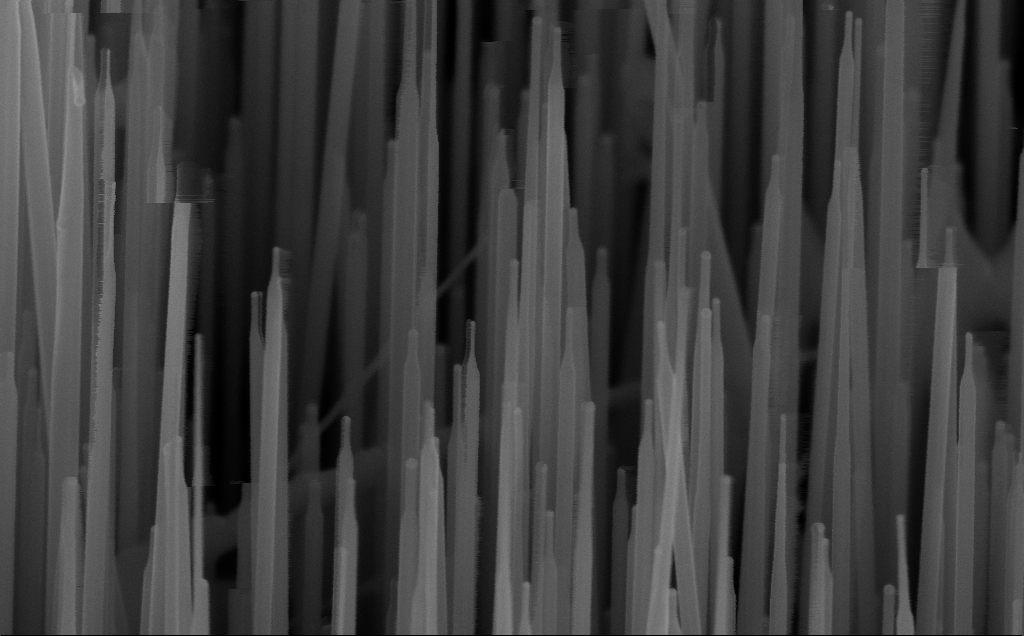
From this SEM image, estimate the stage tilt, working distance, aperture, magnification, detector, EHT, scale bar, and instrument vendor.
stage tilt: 45°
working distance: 7 mm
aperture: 30 µm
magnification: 150 K X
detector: InLens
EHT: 10 kV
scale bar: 200 nm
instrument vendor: Zeiss